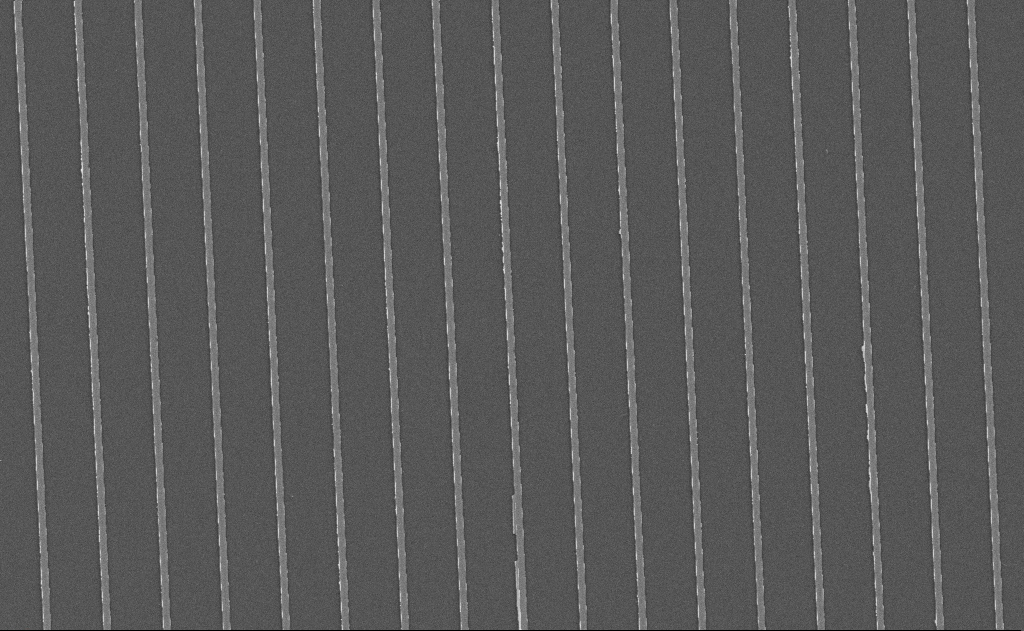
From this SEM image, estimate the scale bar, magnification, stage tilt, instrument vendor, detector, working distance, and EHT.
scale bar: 10000 nm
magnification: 5.41 K X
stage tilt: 0°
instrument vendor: Zeiss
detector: SE2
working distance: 15 mm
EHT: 5 kV